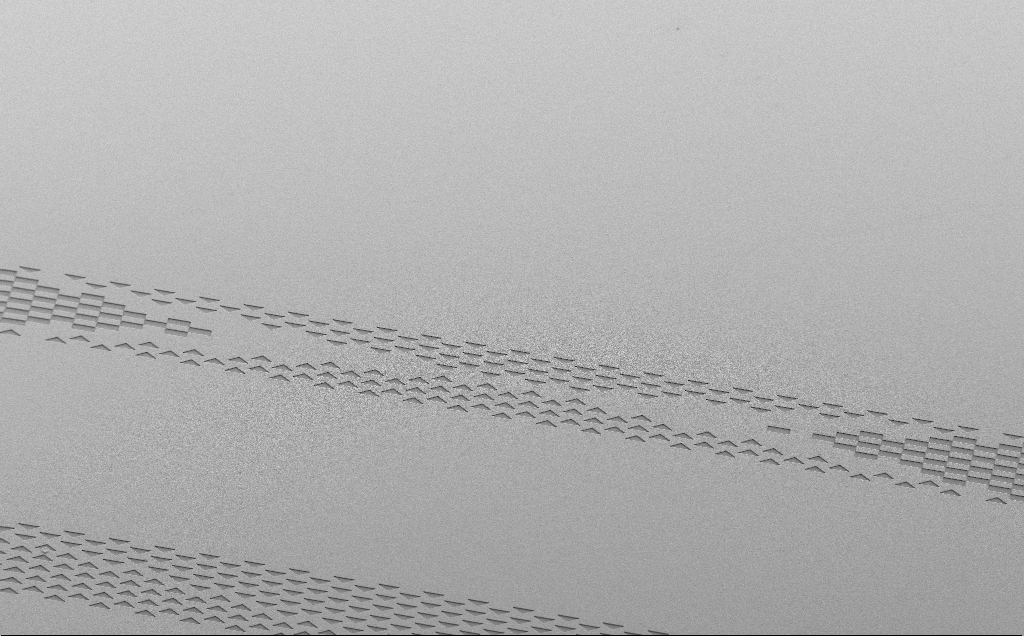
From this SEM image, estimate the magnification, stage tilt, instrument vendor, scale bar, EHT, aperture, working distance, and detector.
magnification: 0.197 K X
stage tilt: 35°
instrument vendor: Zeiss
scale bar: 200000 nm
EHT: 5 kV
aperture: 30 µm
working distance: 13 mm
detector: SE2